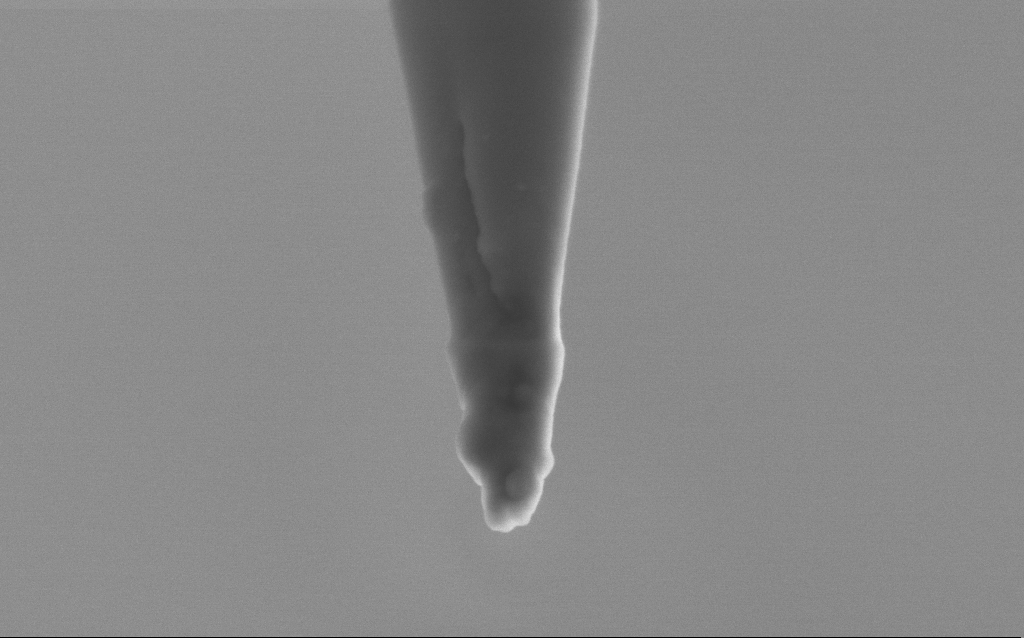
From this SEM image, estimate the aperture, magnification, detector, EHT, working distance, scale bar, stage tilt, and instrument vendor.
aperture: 30 µm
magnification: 100 K X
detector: SE2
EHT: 2.5 kV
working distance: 5 mm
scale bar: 200 nm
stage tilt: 45°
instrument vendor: Zeiss